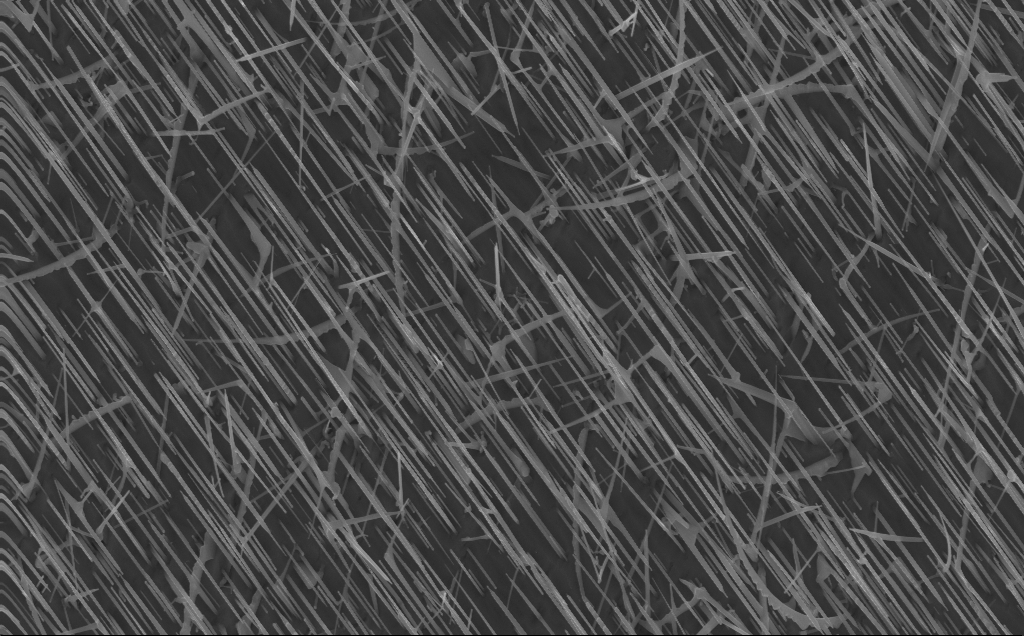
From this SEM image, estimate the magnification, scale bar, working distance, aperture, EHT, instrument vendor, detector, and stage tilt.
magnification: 20 K X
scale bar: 2000 nm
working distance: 4 mm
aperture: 30 µm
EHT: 10 kV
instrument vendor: Zeiss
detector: InLens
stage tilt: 0°